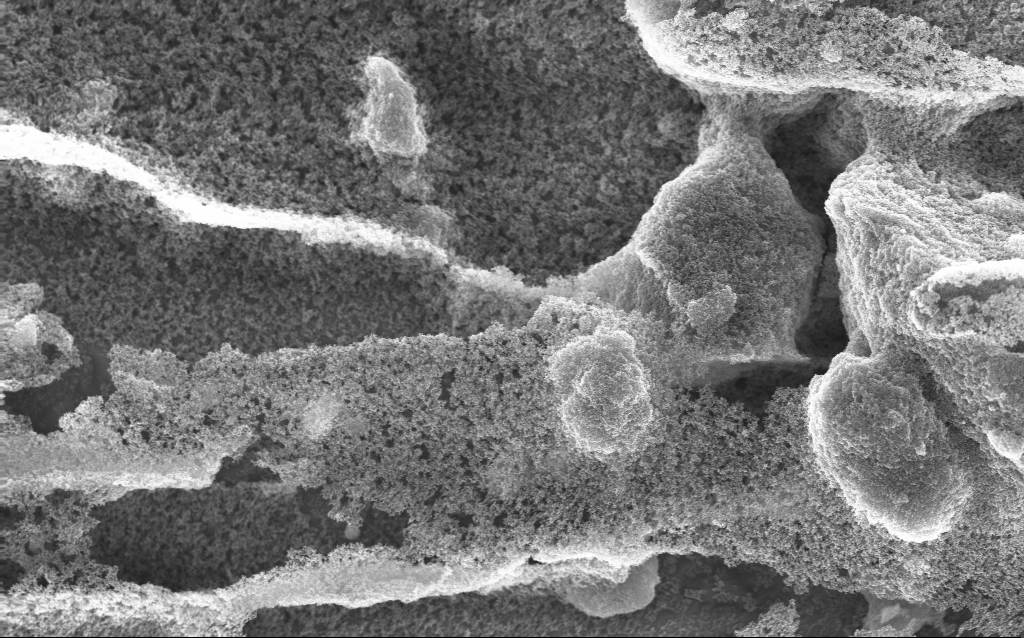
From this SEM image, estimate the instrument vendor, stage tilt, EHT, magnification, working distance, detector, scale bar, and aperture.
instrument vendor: Zeiss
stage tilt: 0°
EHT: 10 kV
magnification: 15.33 K X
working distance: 2.5 mm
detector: InLens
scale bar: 1000 nm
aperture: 30 µm